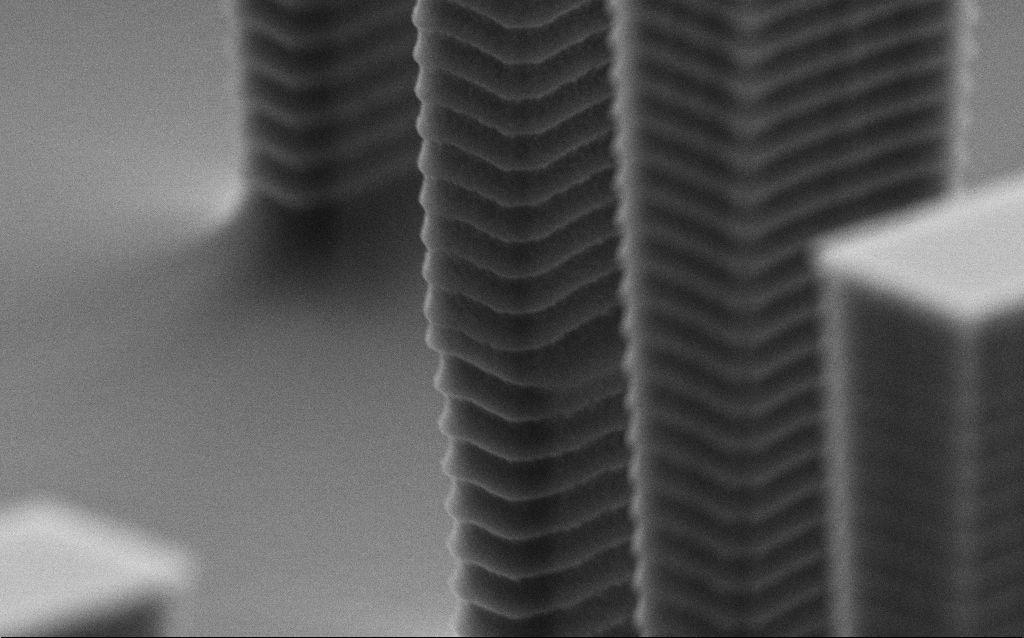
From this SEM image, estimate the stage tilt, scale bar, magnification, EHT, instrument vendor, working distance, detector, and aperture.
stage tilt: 70°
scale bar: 1000 nm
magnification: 43.5 K X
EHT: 6 kV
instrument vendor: Zeiss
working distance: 5.9 mm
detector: SE2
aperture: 30 µm